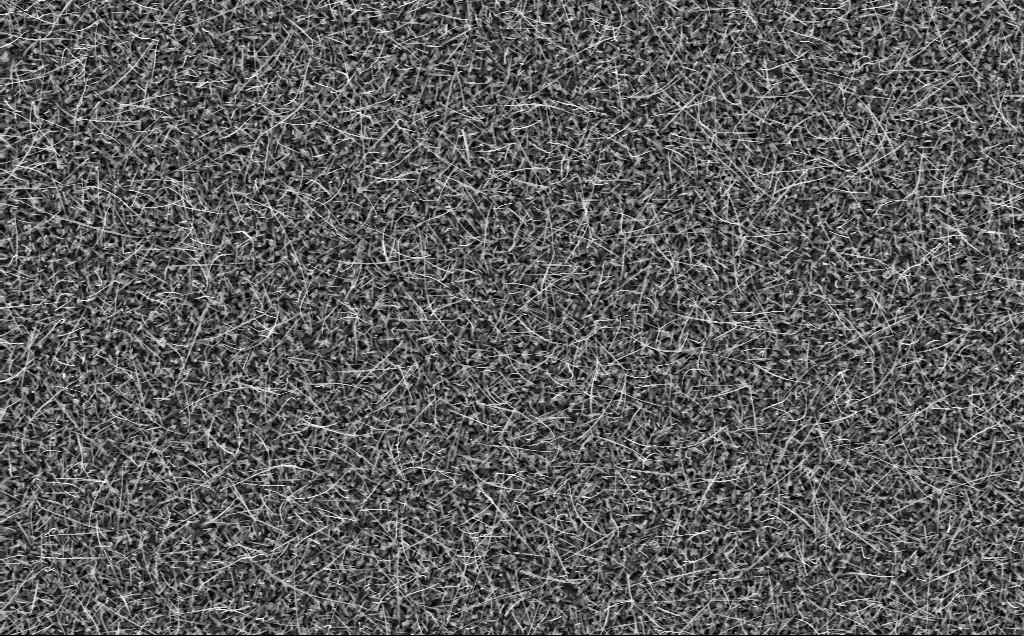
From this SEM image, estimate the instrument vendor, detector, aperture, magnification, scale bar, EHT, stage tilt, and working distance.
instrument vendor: Zeiss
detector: InLens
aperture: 30 µm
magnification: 10 K X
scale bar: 2000 nm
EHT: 10 kV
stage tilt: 0°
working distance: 6 mm